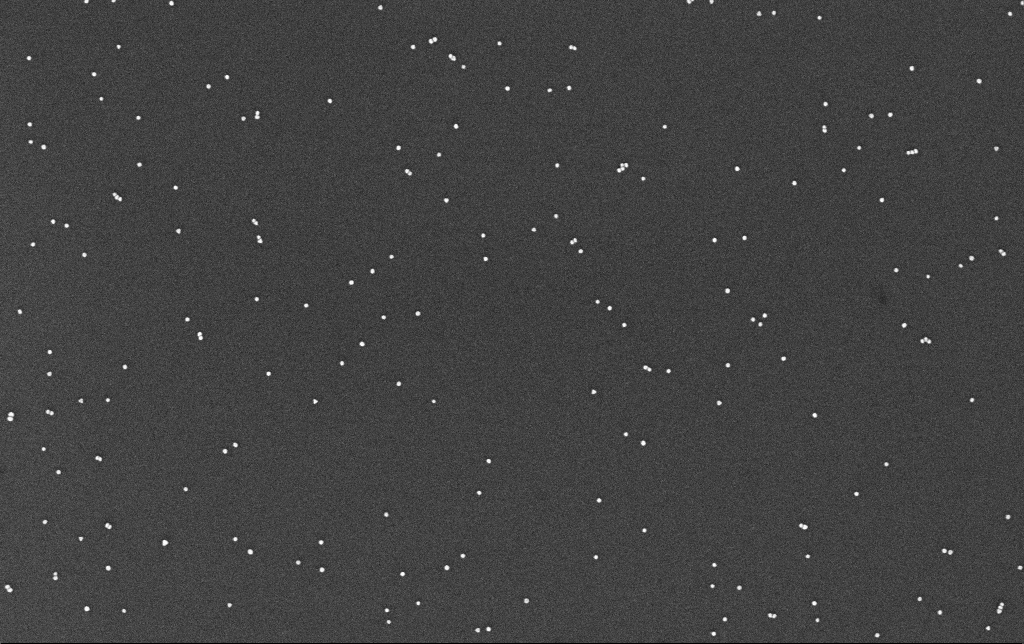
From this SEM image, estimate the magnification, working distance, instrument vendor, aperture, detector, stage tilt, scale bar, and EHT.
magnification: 100 K X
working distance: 3.3 mm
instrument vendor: Zeiss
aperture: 30 µm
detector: InLens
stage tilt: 0°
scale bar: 200 nm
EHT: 10 kV